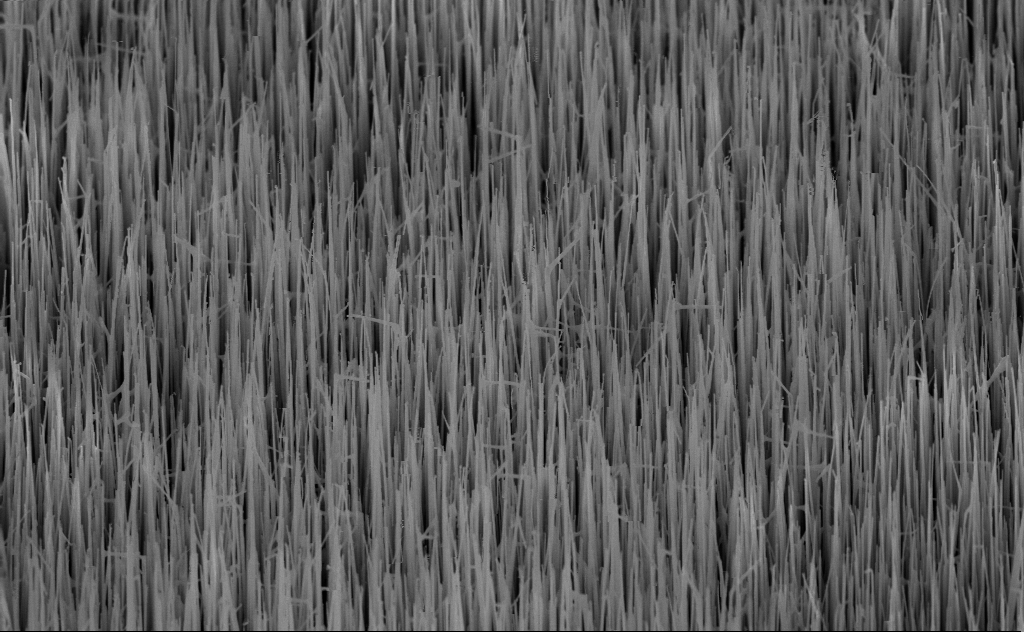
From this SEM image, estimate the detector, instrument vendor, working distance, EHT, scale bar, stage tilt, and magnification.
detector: InLens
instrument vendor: Zeiss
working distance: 6 mm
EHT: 10 kV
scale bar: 2000 nm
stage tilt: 45°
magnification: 20 K X